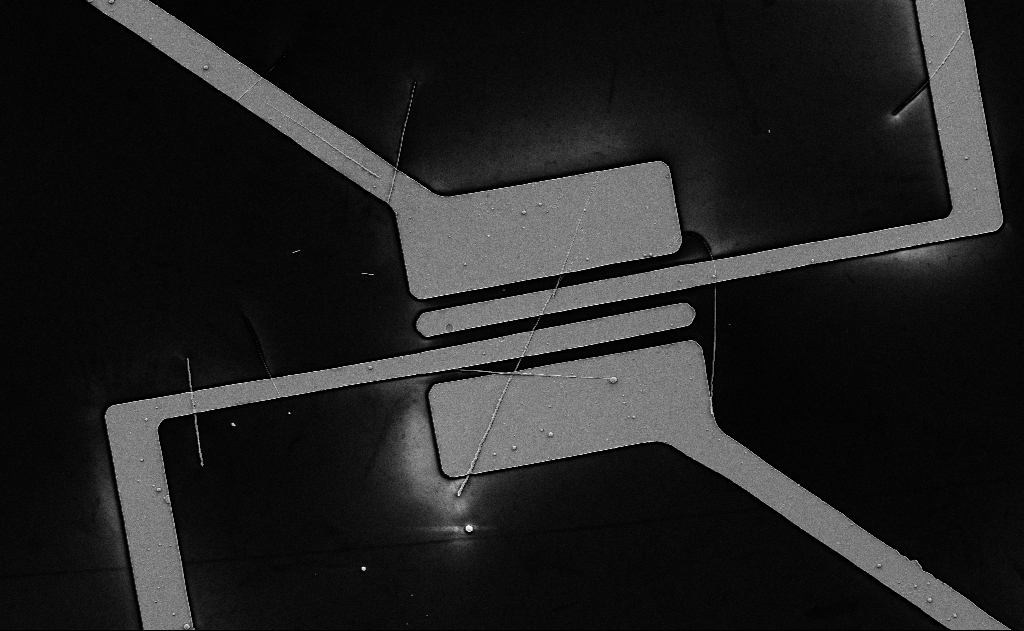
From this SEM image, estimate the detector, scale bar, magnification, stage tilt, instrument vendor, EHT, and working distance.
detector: SE2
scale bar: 10000 nm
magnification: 3.34 K X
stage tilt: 0°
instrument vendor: Zeiss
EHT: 5 kV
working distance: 18 mm